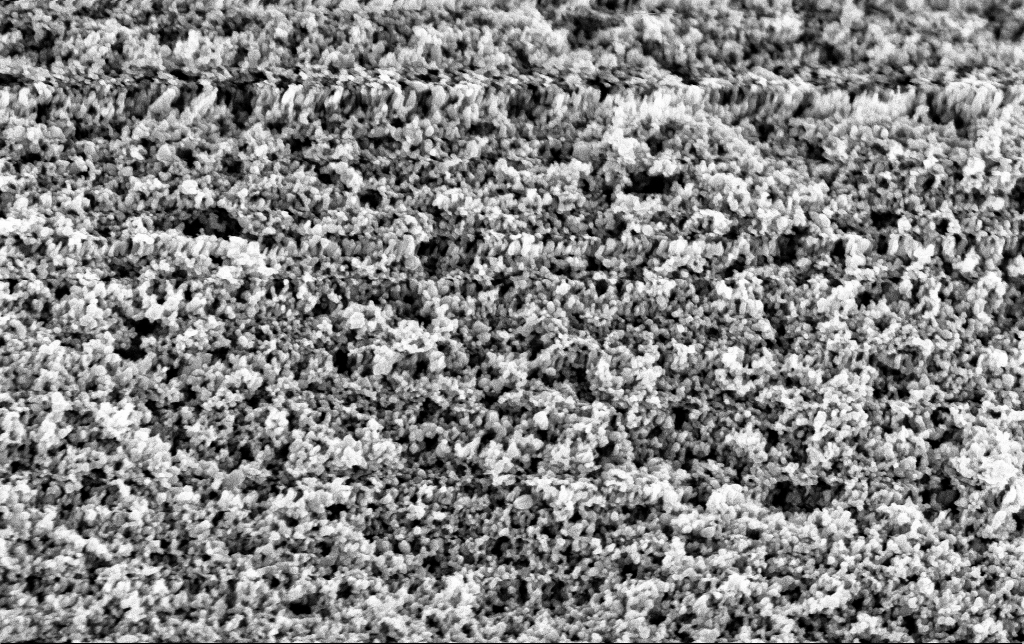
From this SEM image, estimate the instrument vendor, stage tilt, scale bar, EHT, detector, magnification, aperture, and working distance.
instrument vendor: Zeiss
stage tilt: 0°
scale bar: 200 nm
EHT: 3 kV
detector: InLens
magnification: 80 K X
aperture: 30 µm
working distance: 2.8 mm